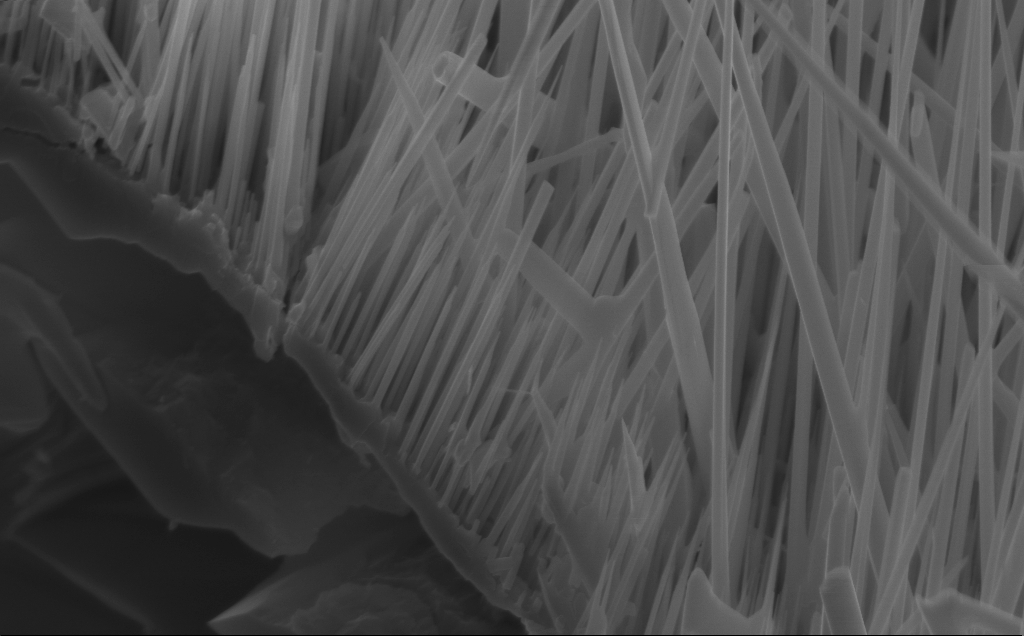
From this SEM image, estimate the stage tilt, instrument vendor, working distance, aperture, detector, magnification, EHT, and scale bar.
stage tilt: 45°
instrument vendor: Zeiss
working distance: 5 mm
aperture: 30 µm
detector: InLens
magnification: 38.1 K X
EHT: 10 kV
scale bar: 1000 nm